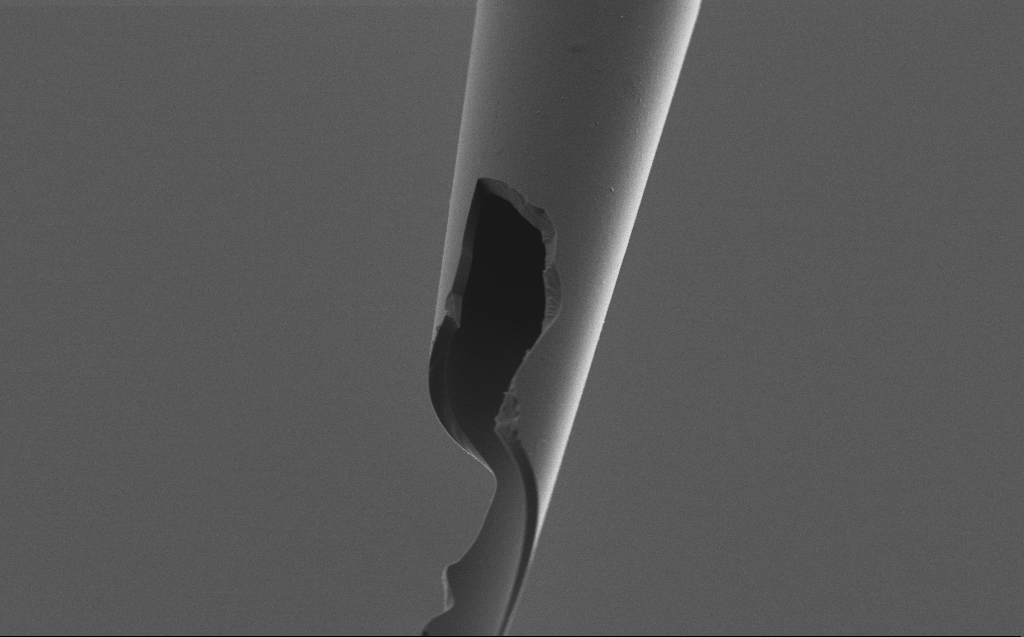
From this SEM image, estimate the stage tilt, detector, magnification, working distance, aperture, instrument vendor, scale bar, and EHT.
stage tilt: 45°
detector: SE2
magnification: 10 K X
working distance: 5 mm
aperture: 30 µm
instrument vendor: Zeiss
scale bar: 2000 nm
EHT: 2 kV